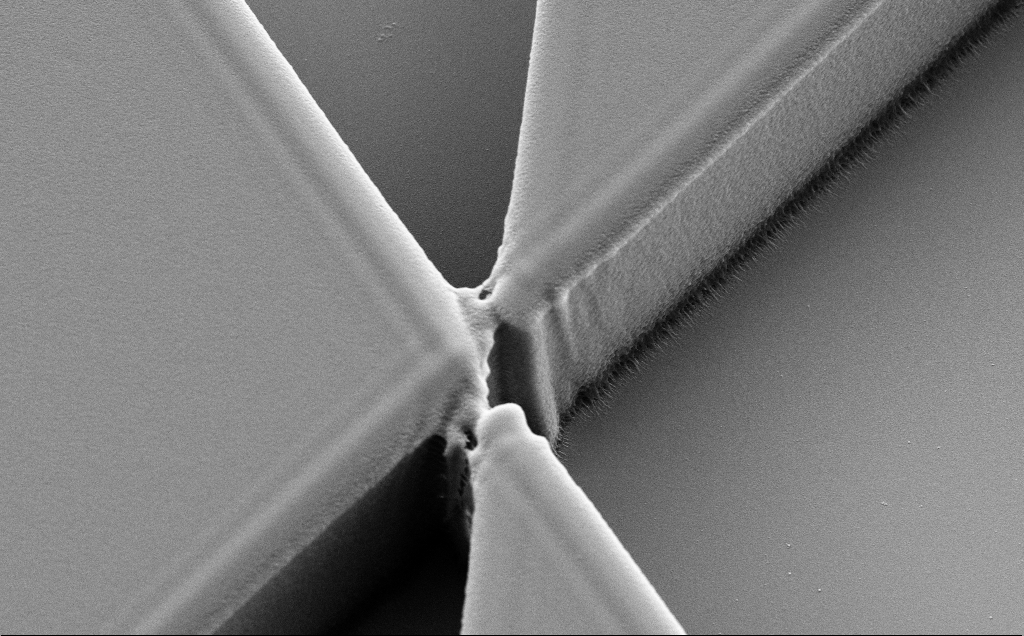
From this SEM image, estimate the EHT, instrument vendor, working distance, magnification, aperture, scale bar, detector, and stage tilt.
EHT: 5 kV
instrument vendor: Zeiss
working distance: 11 mm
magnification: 10.41 K X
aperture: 30 µm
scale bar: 2000 nm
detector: SE2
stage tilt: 30°